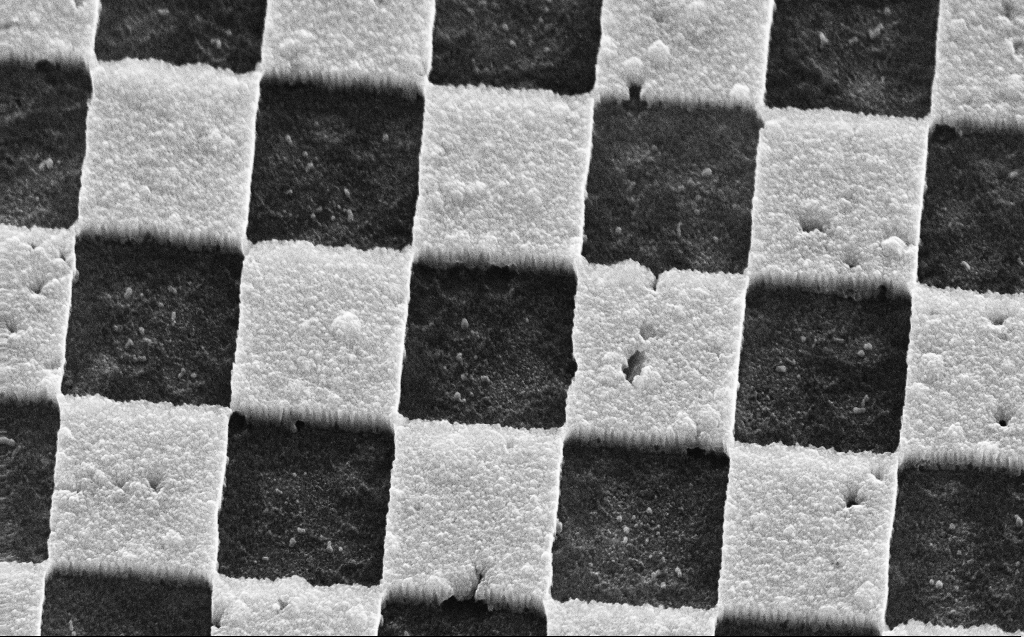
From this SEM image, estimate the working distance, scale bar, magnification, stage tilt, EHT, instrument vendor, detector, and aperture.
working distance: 5 mm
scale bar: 1000 nm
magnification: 62.84 K X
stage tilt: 45°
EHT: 30 kV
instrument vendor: Zeiss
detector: SE2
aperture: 30 µm